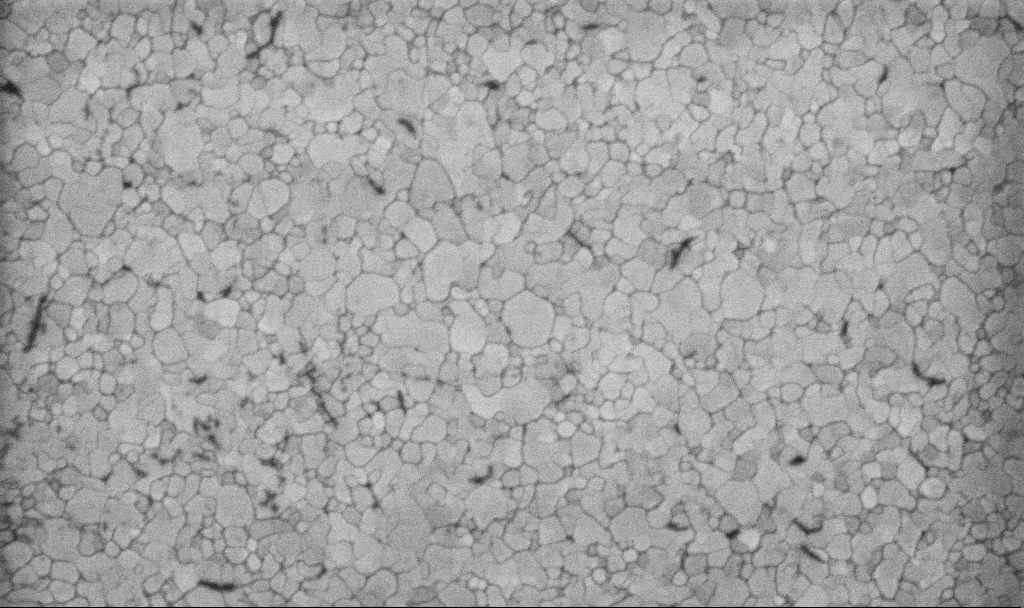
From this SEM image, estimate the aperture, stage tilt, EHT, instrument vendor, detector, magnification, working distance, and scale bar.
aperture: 30 µm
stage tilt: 0°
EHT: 5 kV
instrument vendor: Zeiss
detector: InLens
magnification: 100.15 K X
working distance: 3.1 mm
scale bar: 200 nm